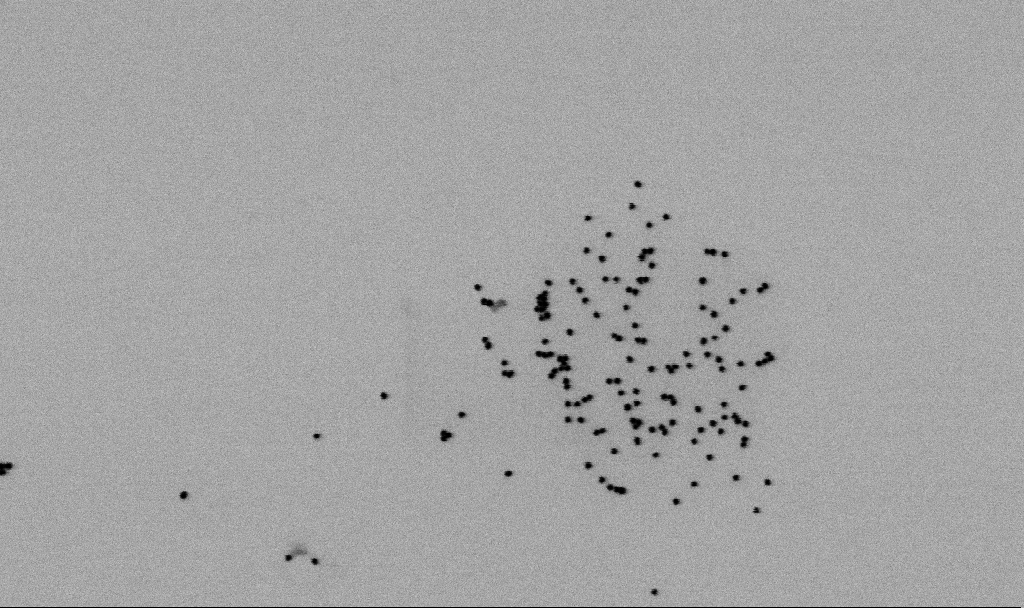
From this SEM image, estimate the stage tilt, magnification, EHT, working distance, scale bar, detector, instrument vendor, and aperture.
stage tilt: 0°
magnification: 104.47 K X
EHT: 2 kV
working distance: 6.5 mm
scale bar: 200 nm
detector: SE2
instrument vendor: Zeiss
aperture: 30 µm